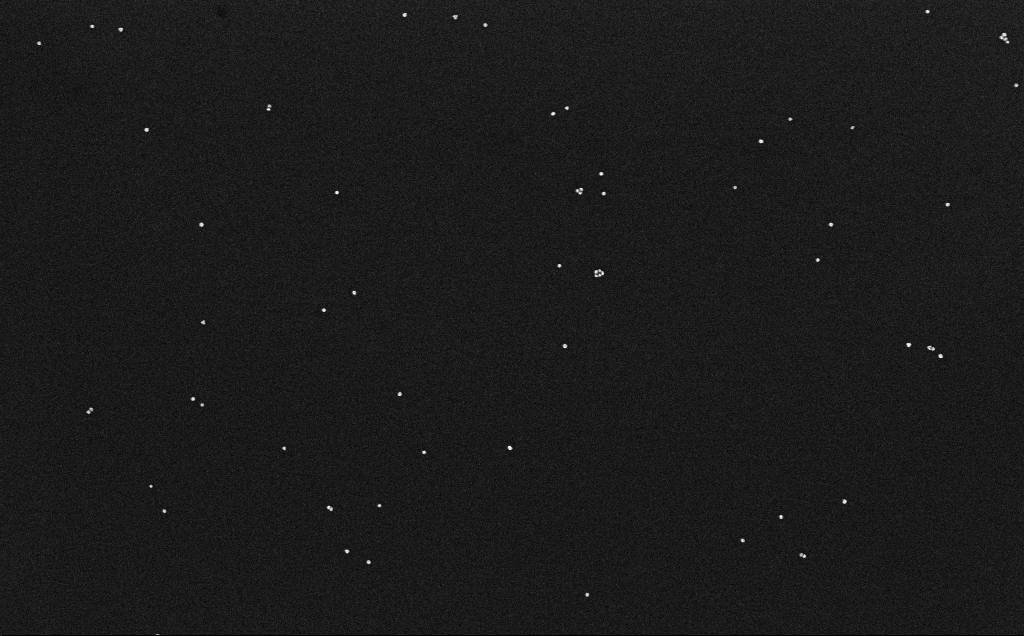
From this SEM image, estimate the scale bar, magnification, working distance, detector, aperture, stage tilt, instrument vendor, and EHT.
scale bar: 200 nm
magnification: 100 K X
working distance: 3.2 mm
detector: InLens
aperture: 30 µm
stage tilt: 0°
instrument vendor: Zeiss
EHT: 10 kV